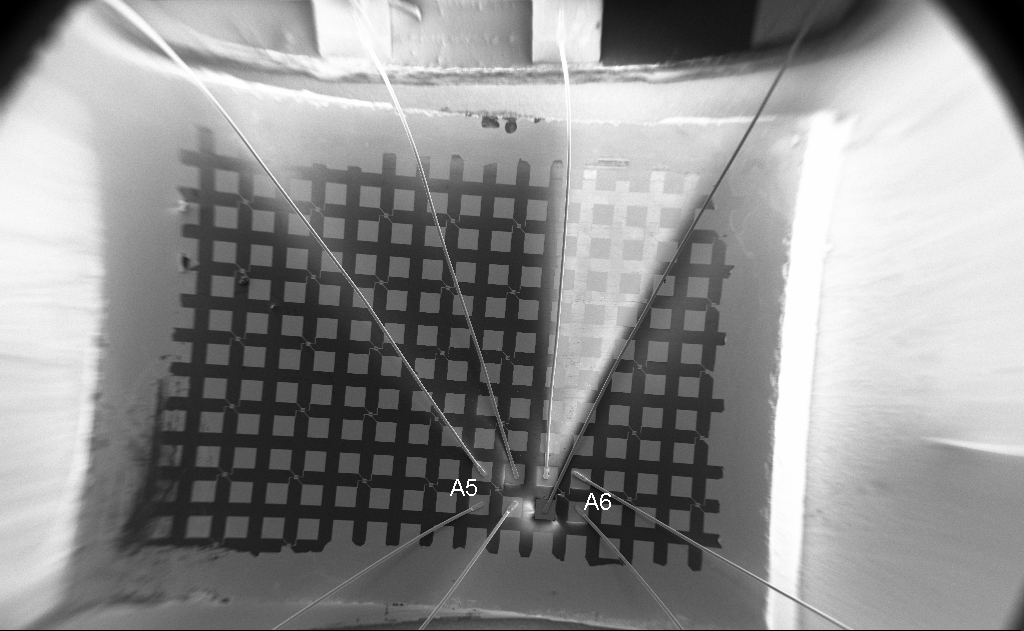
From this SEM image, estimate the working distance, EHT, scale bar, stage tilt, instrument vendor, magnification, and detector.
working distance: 15 mm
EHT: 5 kV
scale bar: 1e+06 nm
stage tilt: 0°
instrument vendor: Zeiss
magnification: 0.051 K X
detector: SE2